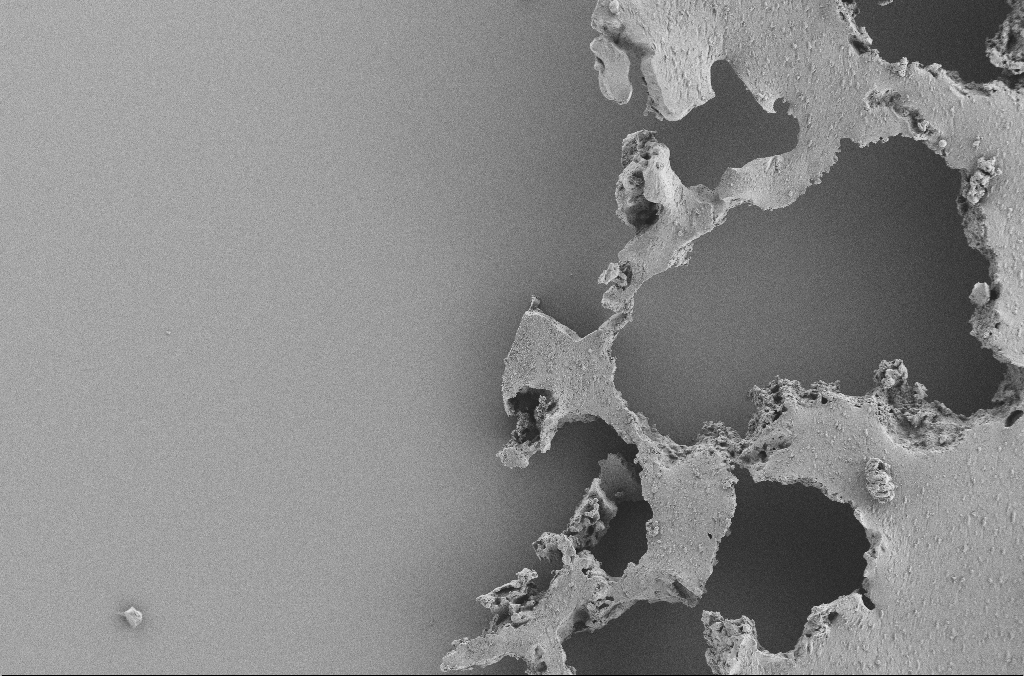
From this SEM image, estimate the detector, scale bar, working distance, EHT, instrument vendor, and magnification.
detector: SE2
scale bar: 100000 nm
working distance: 3.6 mm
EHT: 2 kV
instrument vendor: Zeiss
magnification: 0.25 K X